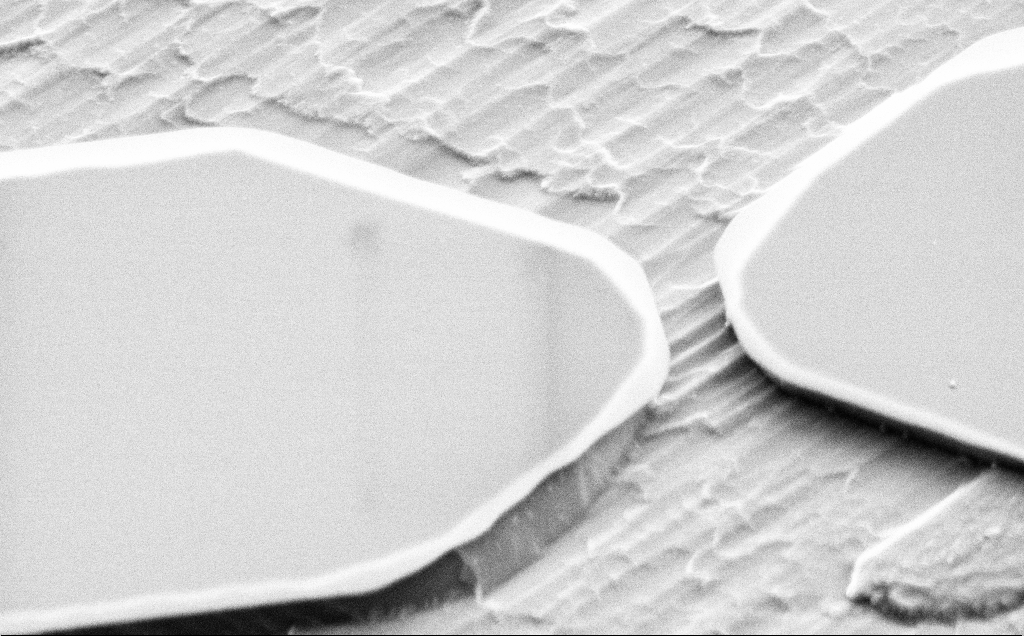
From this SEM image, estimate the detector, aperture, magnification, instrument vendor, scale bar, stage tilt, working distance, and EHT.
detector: SE2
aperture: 30 µm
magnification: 13.79 K X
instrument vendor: Zeiss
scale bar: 2000 nm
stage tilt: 49.4°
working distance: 10 mm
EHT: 5 kV